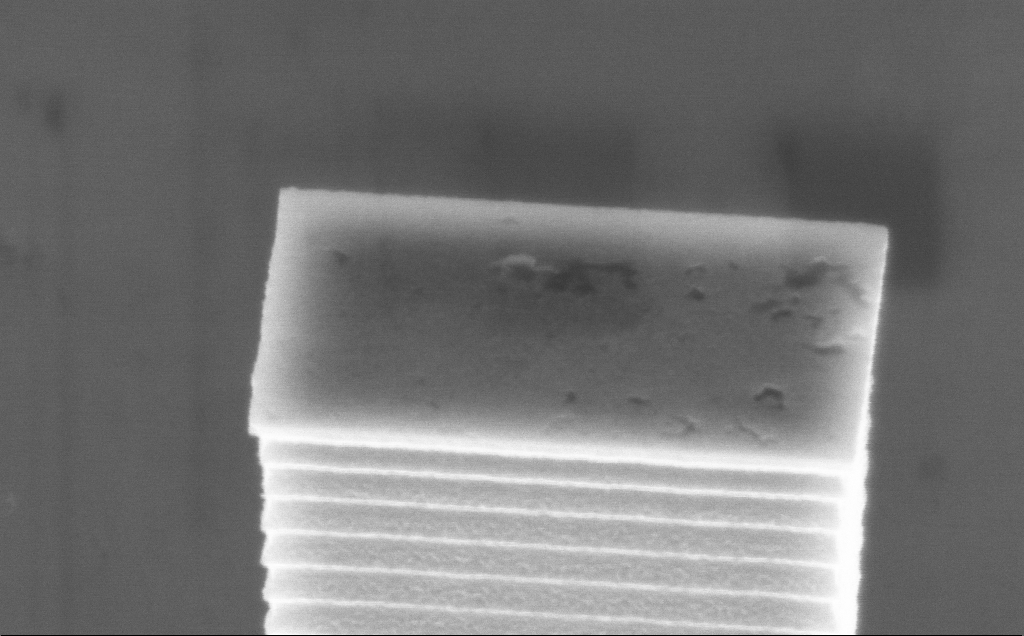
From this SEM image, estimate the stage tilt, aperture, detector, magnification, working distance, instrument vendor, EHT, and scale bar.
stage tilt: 45°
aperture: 30 µm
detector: InLens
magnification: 46.1 K X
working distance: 5 mm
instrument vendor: Zeiss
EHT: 7.5 kV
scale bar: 1000 nm